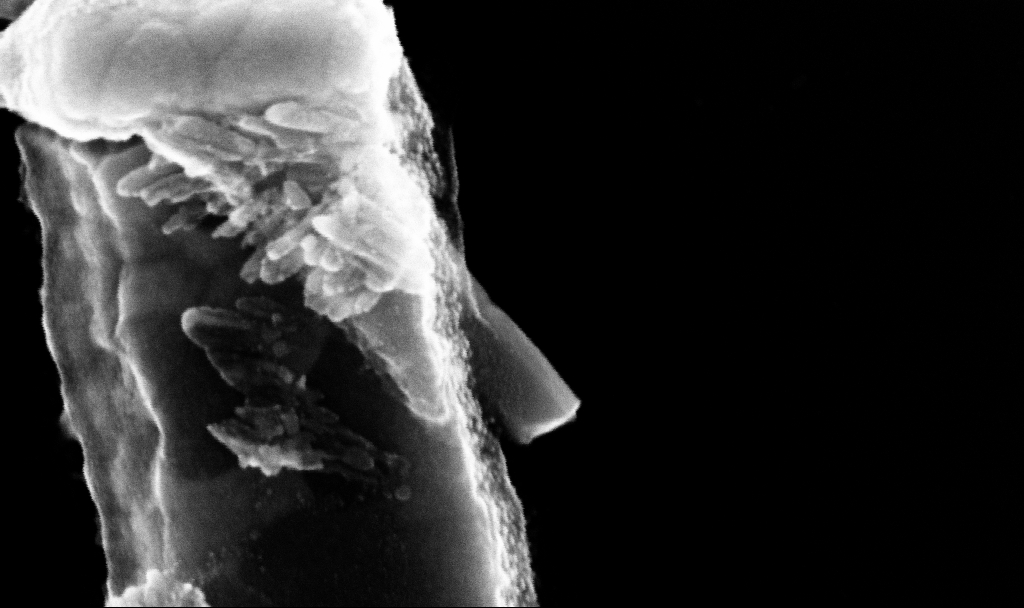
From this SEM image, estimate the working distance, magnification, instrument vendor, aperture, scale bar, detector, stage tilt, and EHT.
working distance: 7.7 mm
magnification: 246.42 K X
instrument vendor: Zeiss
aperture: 30 µm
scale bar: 200 nm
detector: InLens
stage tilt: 0°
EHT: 10 kV